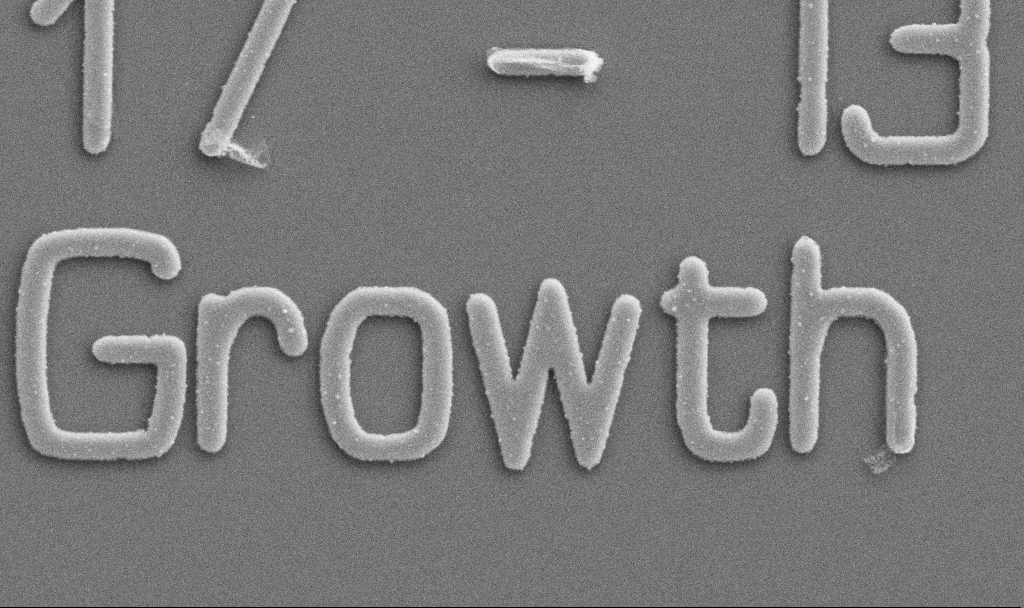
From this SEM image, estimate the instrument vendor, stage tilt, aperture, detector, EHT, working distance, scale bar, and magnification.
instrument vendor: Zeiss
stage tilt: -0°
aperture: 30 µm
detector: SE2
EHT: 5 kV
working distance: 10.7 mm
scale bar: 1000 nm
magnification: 20 K X